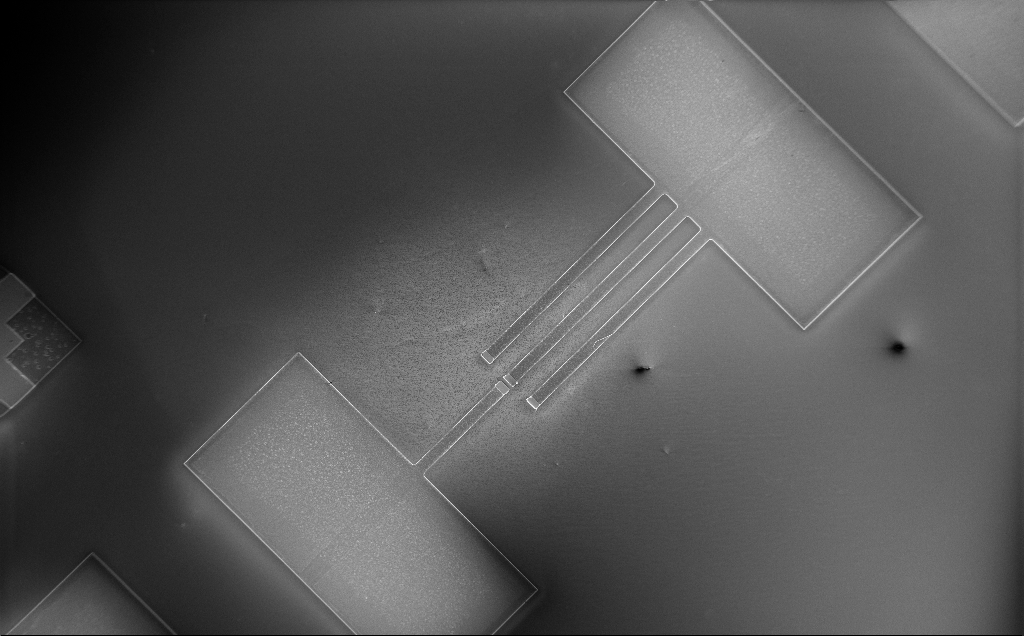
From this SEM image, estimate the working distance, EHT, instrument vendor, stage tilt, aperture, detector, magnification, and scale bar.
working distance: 10 mm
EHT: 2 kV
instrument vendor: Zeiss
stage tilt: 0°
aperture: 30 µm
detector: InLens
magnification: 0.296 K X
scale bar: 100000 nm